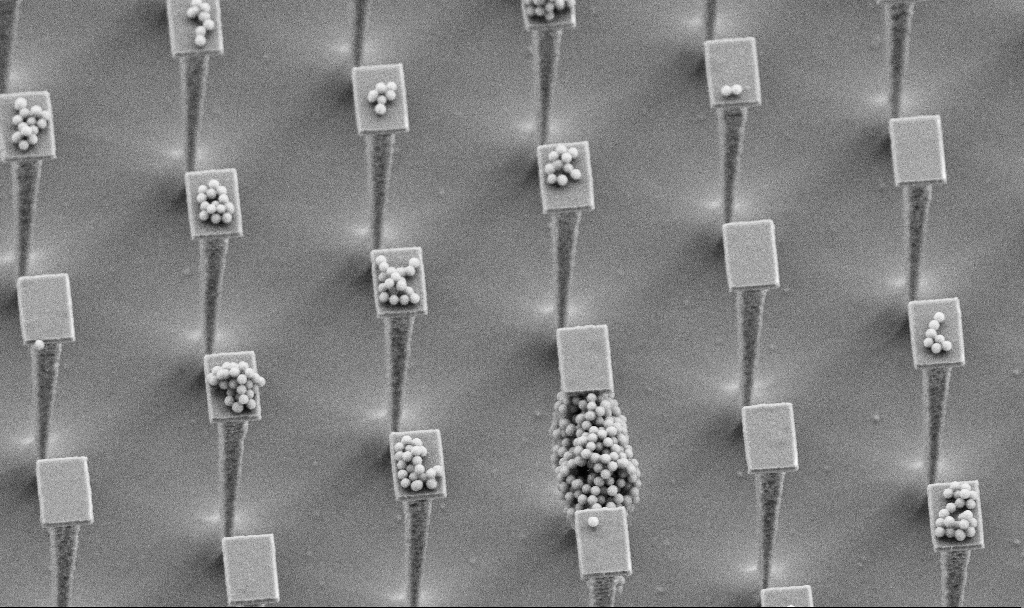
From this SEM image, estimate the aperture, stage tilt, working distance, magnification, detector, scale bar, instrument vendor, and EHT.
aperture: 30 µm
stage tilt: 30°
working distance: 4.5 mm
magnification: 6.47 K X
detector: SE2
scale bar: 10000 nm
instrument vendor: Zeiss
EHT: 5 kV